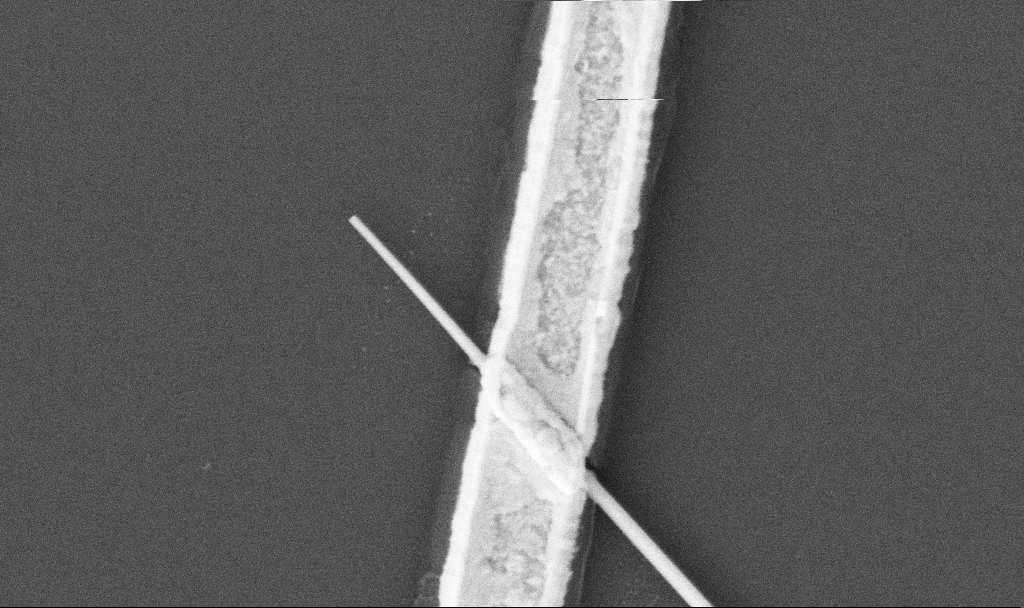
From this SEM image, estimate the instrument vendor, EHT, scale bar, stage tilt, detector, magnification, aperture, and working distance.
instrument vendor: Zeiss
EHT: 5 kV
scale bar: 1000 nm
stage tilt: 0°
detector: SE2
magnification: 60 K X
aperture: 30 µm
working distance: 10.8 mm